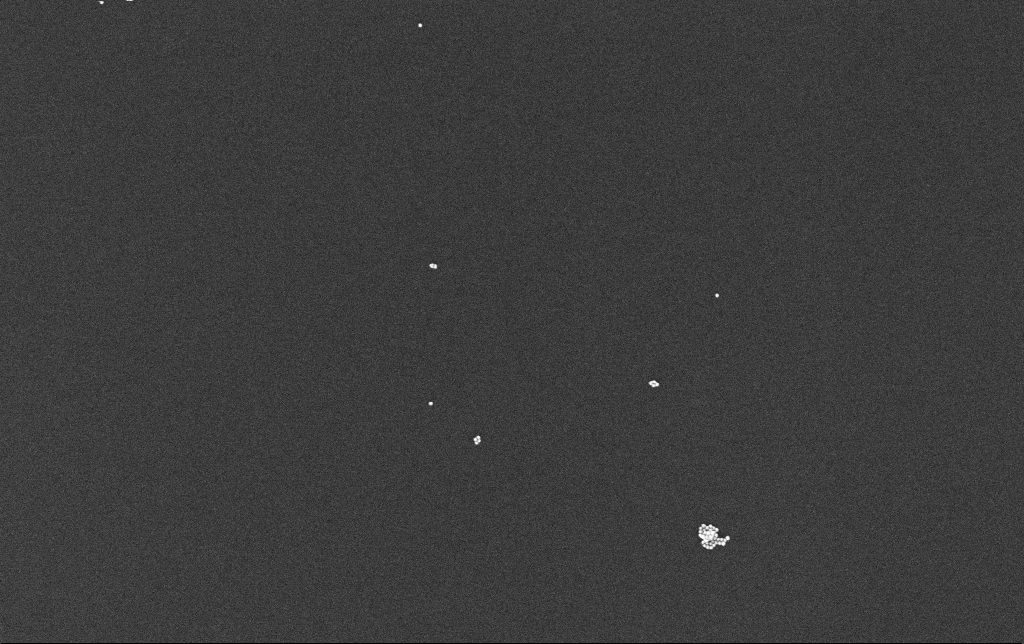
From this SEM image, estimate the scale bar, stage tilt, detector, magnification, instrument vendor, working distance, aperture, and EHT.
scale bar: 200 nm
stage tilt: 0°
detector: InLens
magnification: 100 K X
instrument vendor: Zeiss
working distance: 3.4 mm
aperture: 30 µm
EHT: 10 kV